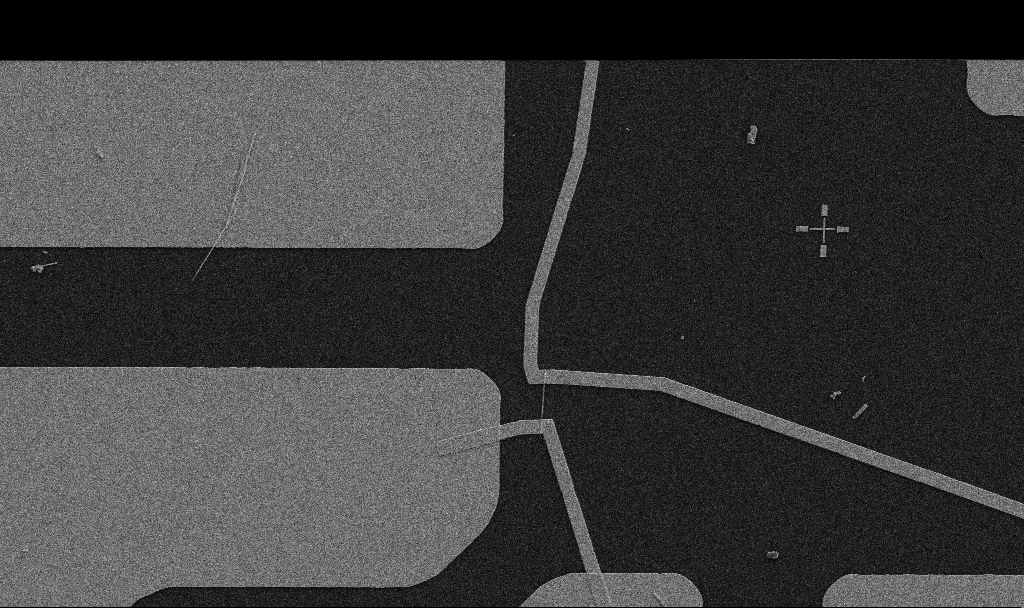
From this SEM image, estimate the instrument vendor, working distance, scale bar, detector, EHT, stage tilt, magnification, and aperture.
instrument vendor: Zeiss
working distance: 10.7 mm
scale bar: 10000 nm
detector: SE2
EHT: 5 kV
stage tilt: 0°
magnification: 5 K X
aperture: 30 µm